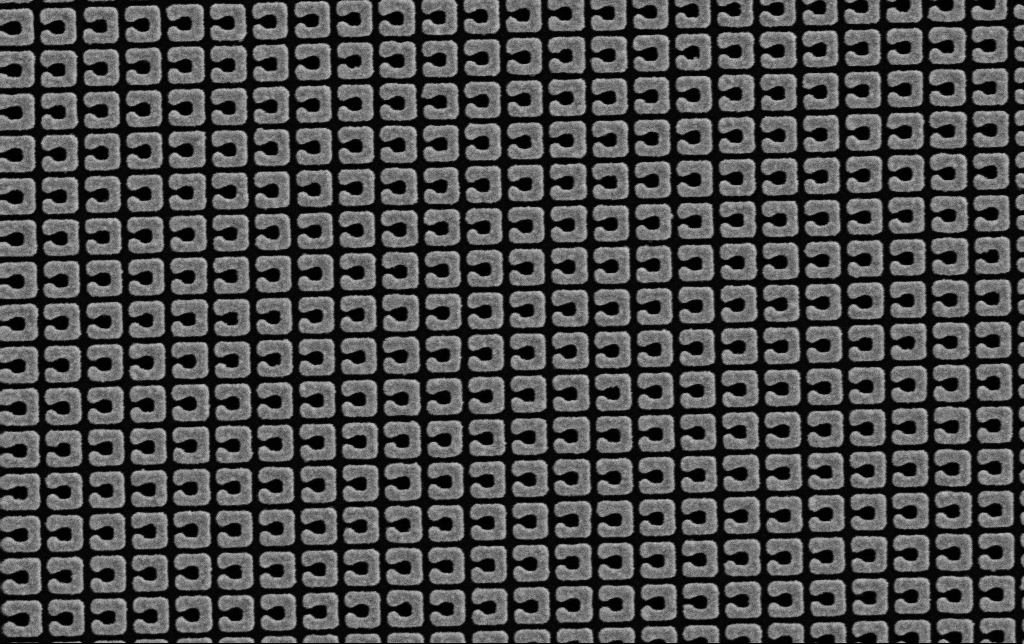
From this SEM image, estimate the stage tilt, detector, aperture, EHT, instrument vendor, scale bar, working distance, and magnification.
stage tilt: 0°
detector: SE2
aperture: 30 µm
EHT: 5 kV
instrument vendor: Zeiss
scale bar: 1000 nm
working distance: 4.2 mm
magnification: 33.97 K X